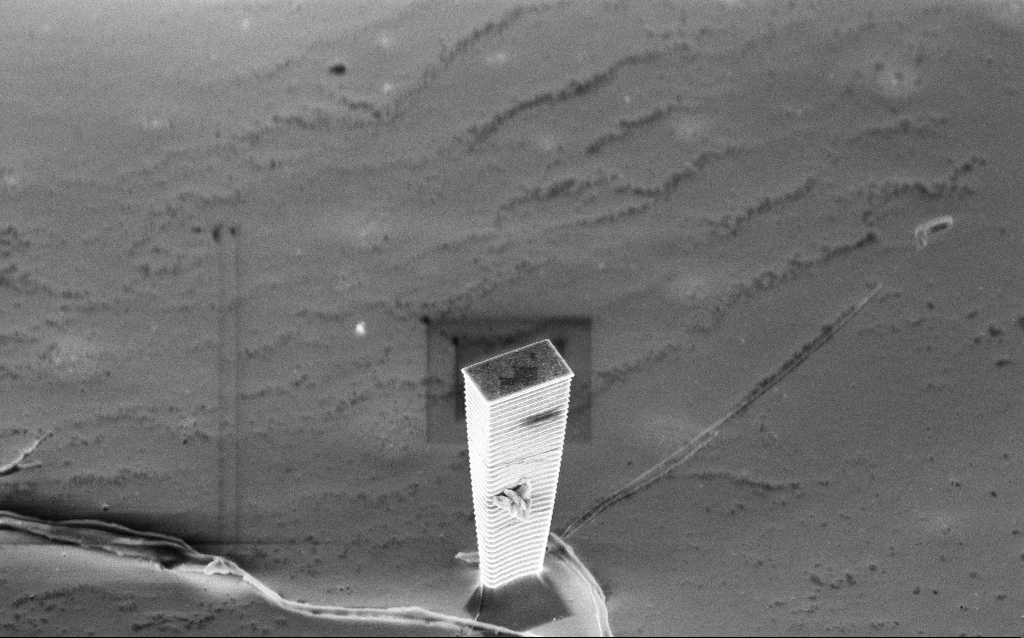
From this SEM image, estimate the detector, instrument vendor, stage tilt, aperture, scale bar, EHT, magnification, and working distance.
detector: InLens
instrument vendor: Zeiss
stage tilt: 45°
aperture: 30 µm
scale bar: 2000 nm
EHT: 5 kV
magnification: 7.42 K X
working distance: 4 mm